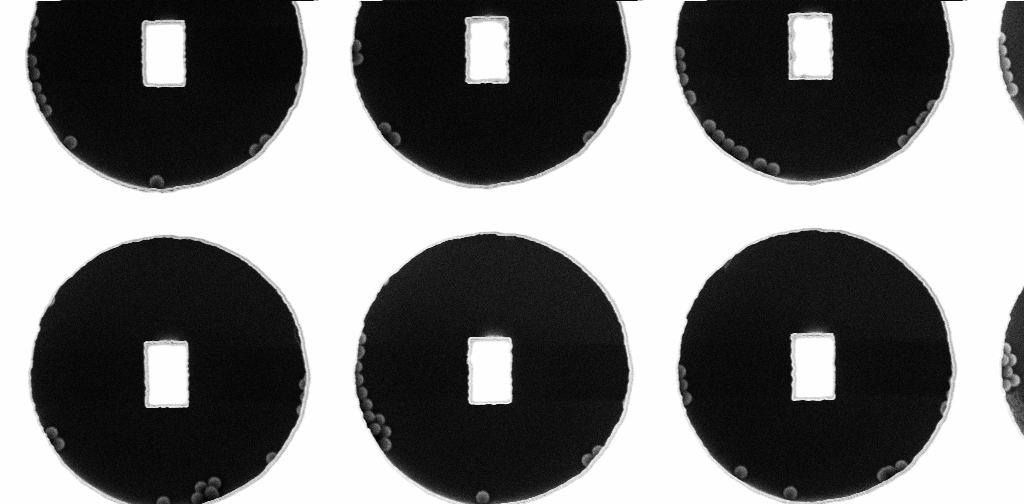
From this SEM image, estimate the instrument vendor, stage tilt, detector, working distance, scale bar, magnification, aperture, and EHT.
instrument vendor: Zeiss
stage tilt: -0°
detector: InLens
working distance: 3.3 mm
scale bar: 2000 nm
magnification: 8.5 K X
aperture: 30 µm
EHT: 5 kV